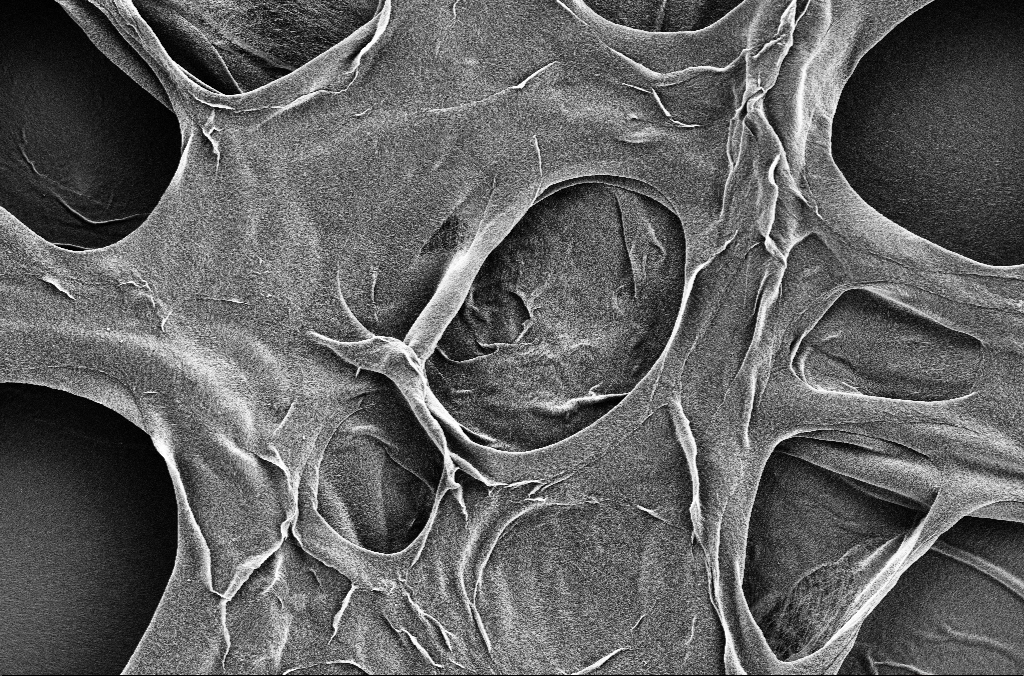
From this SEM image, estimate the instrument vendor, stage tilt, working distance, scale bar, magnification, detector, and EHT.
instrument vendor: Zeiss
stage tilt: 0°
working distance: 5.7 mm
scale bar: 10000 nm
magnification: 2.5 K X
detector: SE2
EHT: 1.8 kV